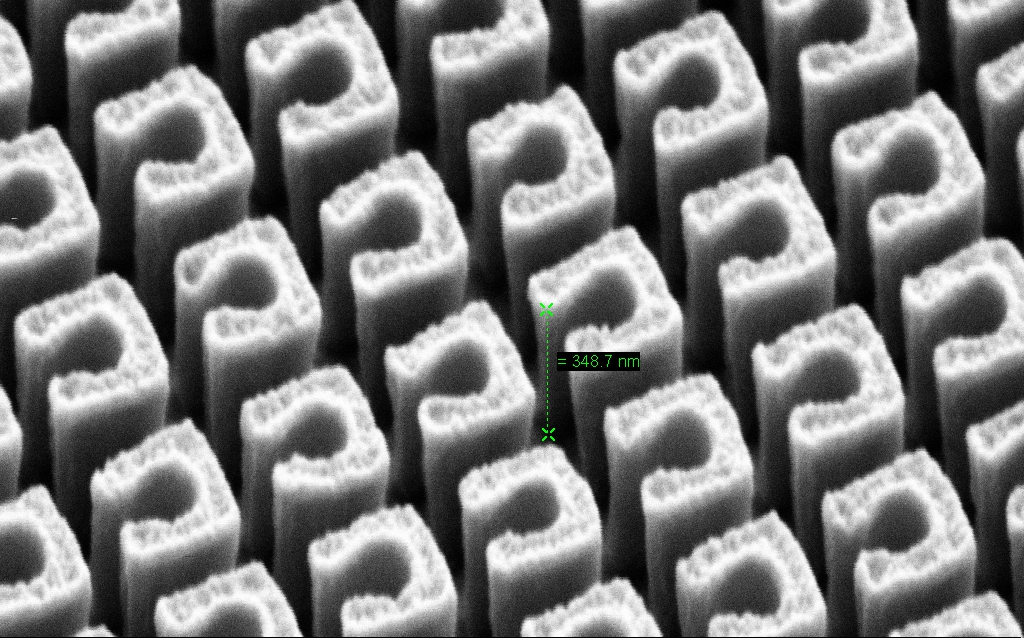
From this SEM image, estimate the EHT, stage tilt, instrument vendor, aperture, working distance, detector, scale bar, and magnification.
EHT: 3 kV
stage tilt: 45°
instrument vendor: Zeiss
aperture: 30 µm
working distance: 7.3 mm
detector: SE2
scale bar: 200 nm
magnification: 131.63 K X